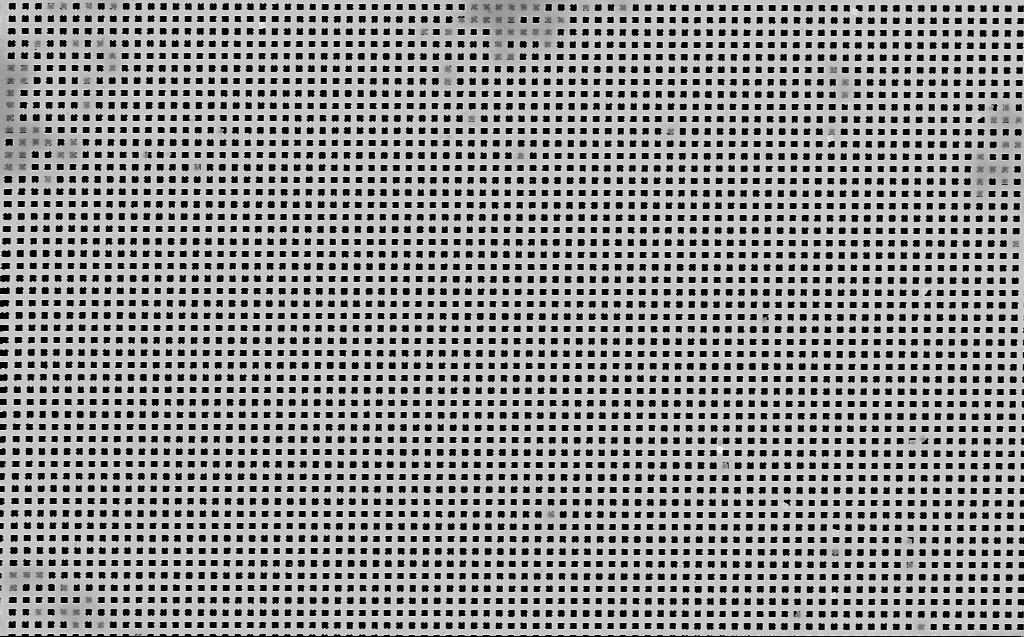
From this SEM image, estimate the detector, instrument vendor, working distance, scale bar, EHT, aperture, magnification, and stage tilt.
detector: InLens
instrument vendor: Zeiss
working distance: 7 mm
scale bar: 2000 nm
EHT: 10 kV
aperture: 30 µm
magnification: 9.22 K X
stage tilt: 0°